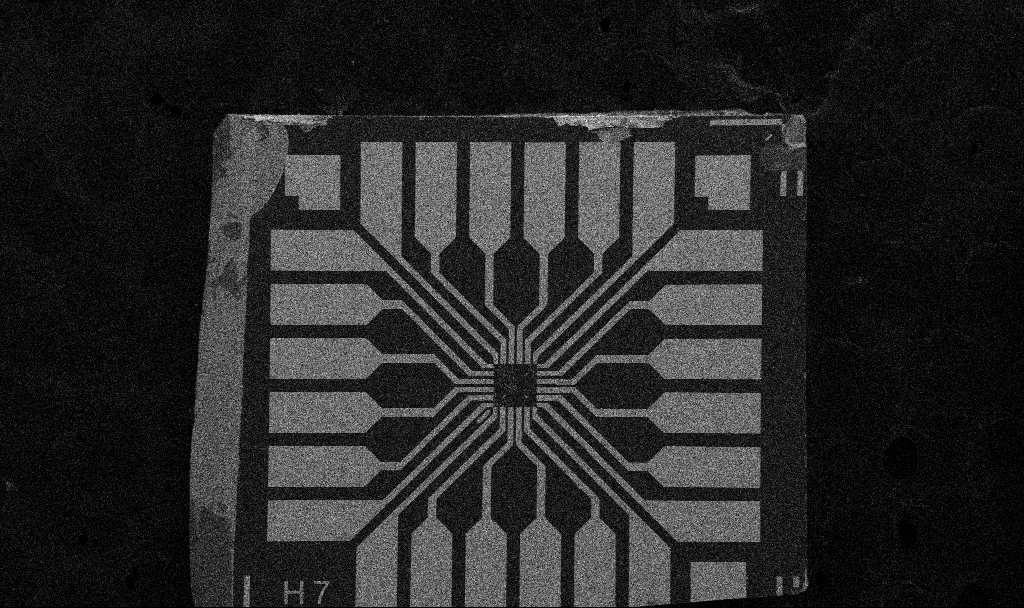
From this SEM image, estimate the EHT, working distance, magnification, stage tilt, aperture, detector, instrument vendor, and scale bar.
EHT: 5 kV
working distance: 10.7 mm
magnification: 0.1 K X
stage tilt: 0°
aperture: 30 µm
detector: SE2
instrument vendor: Zeiss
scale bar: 200000 nm